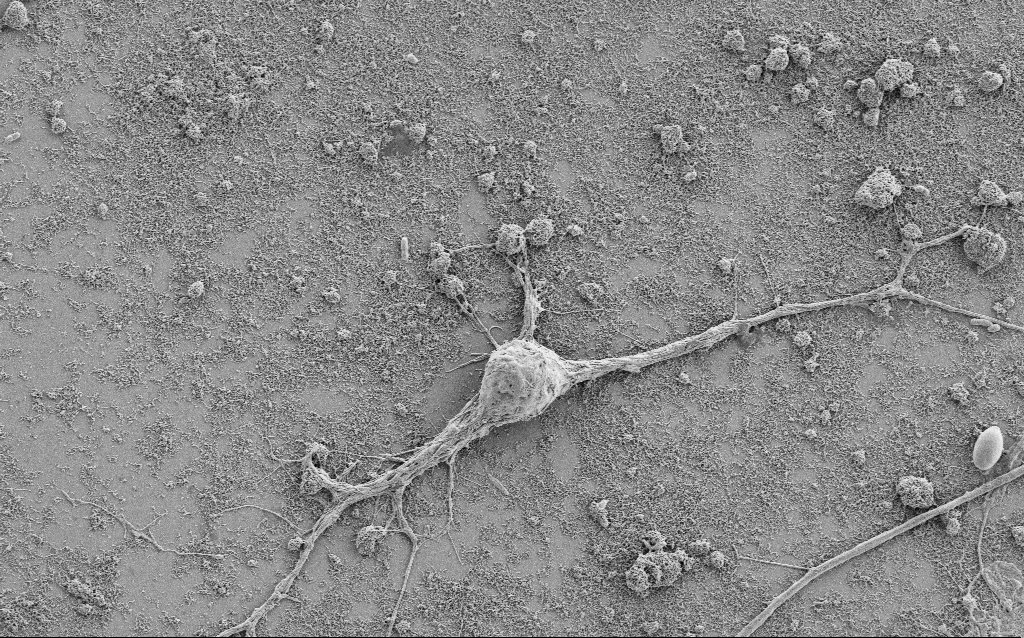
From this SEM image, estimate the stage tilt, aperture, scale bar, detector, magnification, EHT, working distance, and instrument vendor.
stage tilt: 0°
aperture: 30 µm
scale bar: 10000 nm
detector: SE2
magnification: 4 K X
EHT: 1.5 kV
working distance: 6.8 mm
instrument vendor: Zeiss